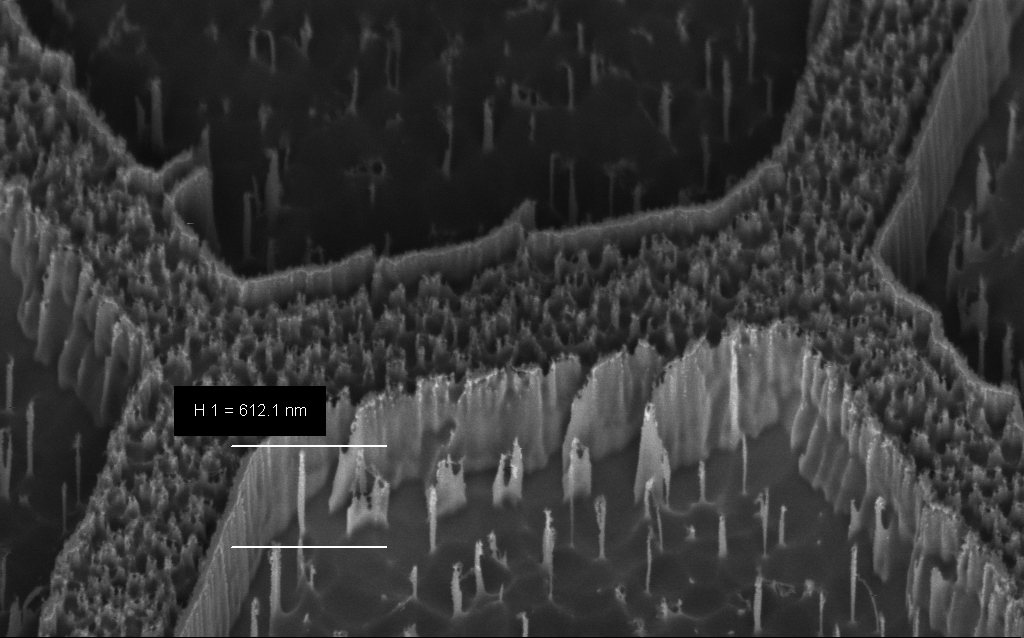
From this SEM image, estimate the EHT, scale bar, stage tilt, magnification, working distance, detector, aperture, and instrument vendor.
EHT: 2 kV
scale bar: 1000 nm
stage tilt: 45°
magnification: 60.59 K X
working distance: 7 mm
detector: InLens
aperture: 30 µm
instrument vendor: Zeiss